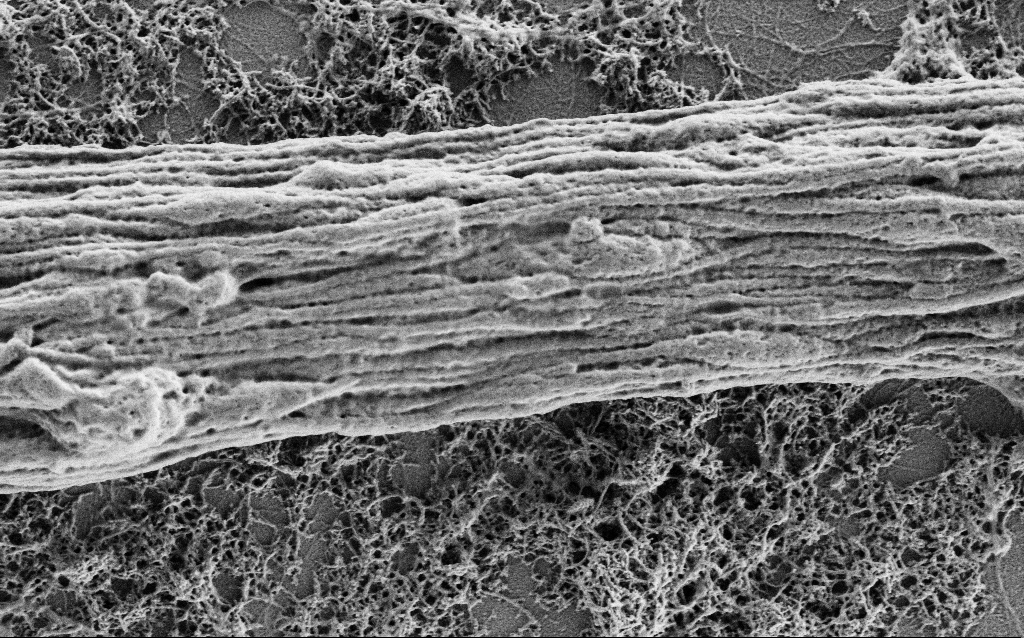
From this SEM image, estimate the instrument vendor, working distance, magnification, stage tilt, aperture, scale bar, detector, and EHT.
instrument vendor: Zeiss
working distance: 4 mm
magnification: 25 K X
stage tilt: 0°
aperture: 30 µm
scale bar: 1000 nm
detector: SE2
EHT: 1 kV